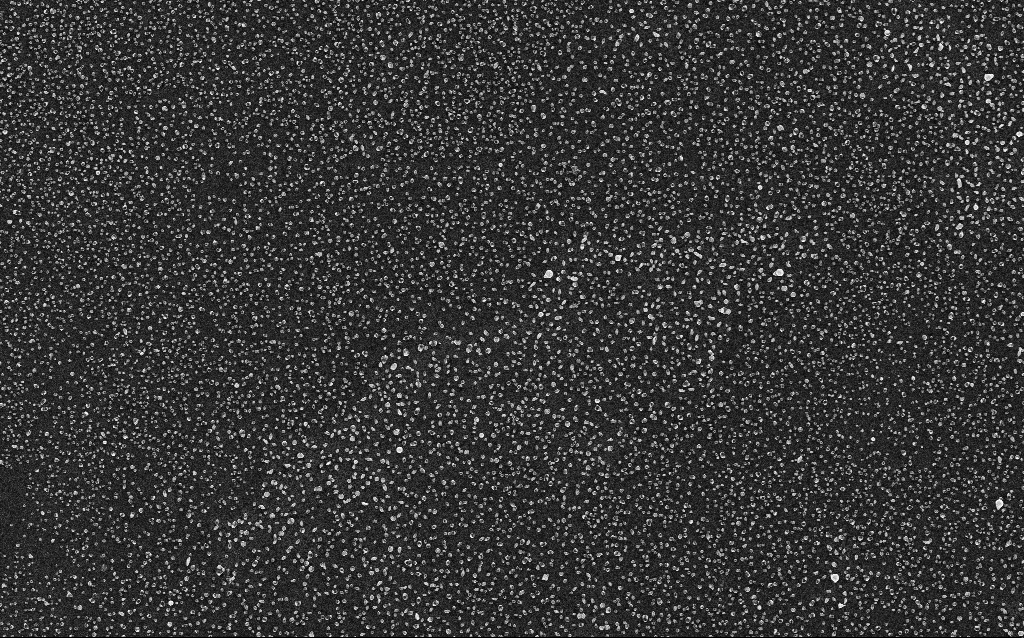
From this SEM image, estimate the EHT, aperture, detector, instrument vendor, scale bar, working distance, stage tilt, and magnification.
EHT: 5 kV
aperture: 30 µm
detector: InLens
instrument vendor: Zeiss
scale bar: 2000 nm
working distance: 2.6 mm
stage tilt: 0°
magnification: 10 K X